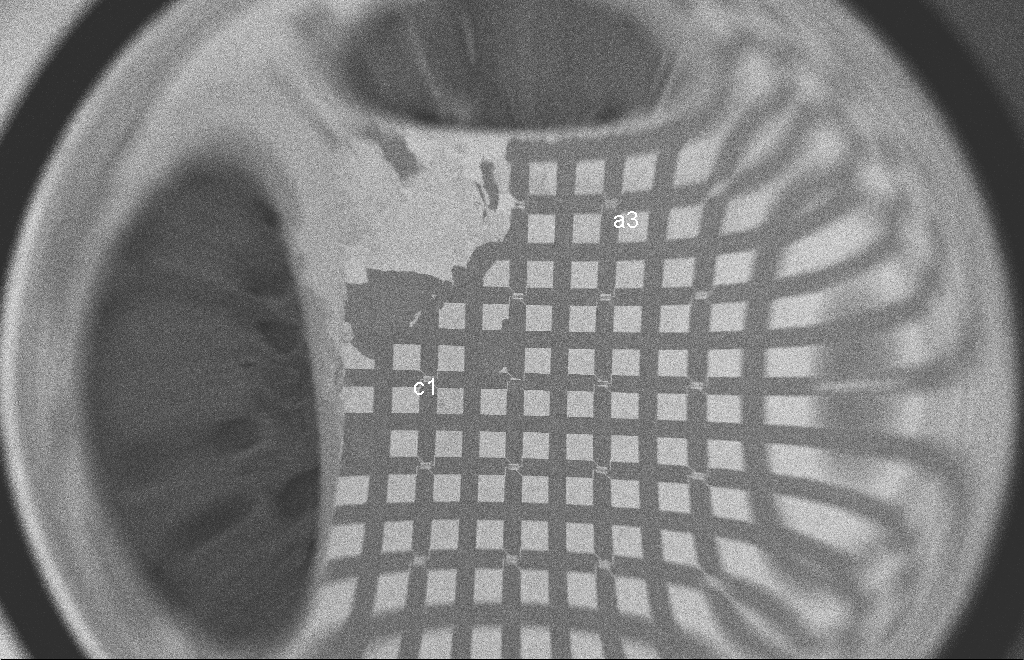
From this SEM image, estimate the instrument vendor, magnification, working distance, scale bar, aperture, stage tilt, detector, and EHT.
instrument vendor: Zeiss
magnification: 0.064 K X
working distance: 8 mm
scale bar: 200000 nm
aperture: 20 µm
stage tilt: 0°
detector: SE2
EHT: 2 kV